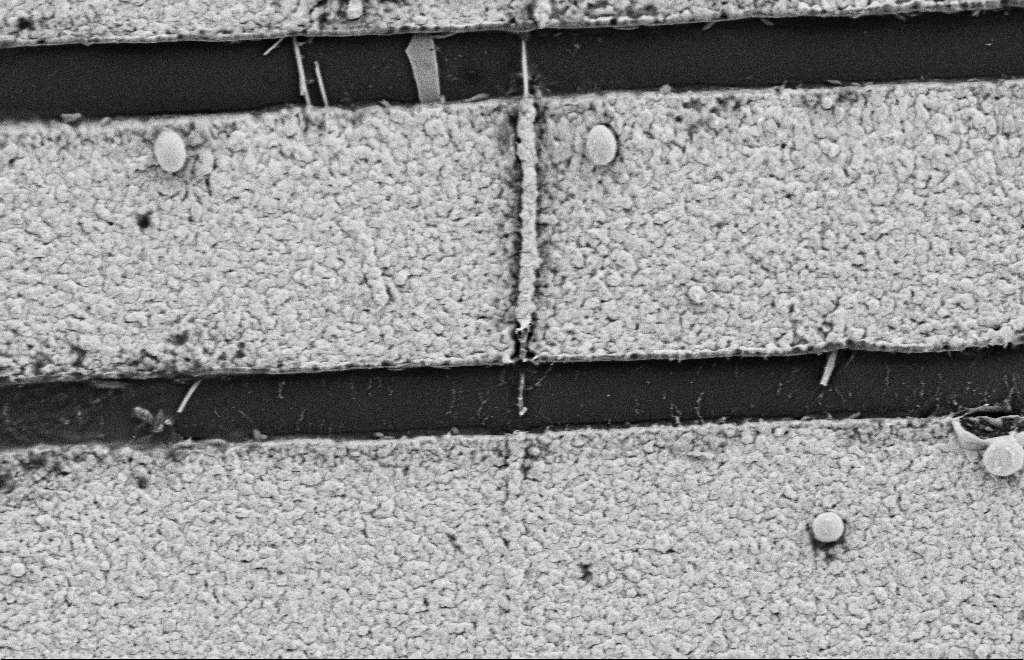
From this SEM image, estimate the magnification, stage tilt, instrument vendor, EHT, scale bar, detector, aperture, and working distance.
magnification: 29.86 K X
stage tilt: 0°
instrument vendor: Zeiss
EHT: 2 kV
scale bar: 1000 nm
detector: SE2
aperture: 20 µm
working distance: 9 mm